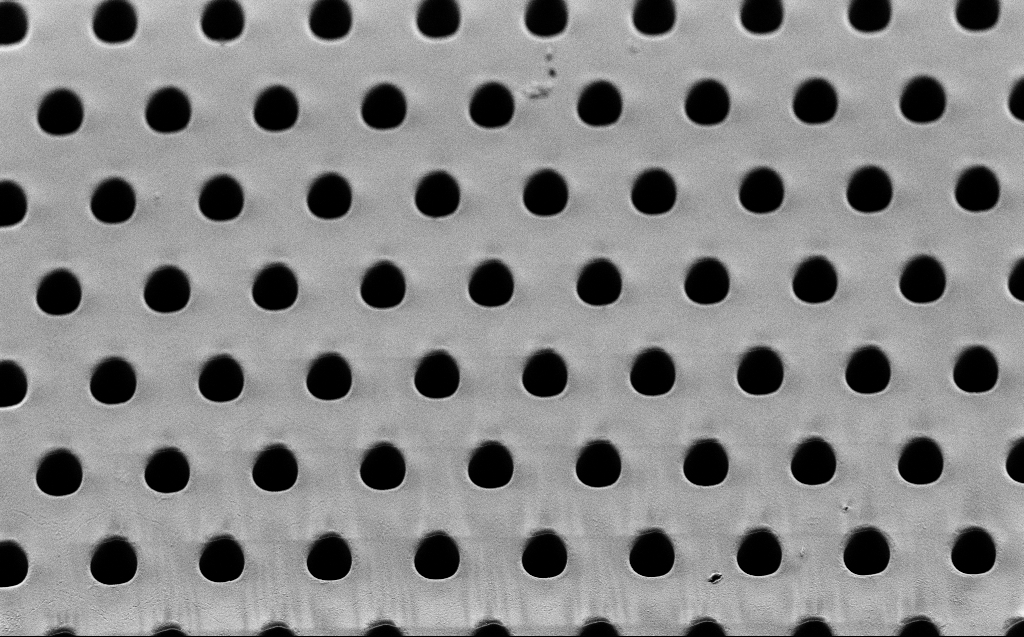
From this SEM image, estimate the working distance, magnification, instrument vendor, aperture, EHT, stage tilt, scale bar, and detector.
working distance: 6 mm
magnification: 2.29 K X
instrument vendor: Zeiss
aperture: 30 µm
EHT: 2 kV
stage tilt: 45°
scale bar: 20000 nm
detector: SE2